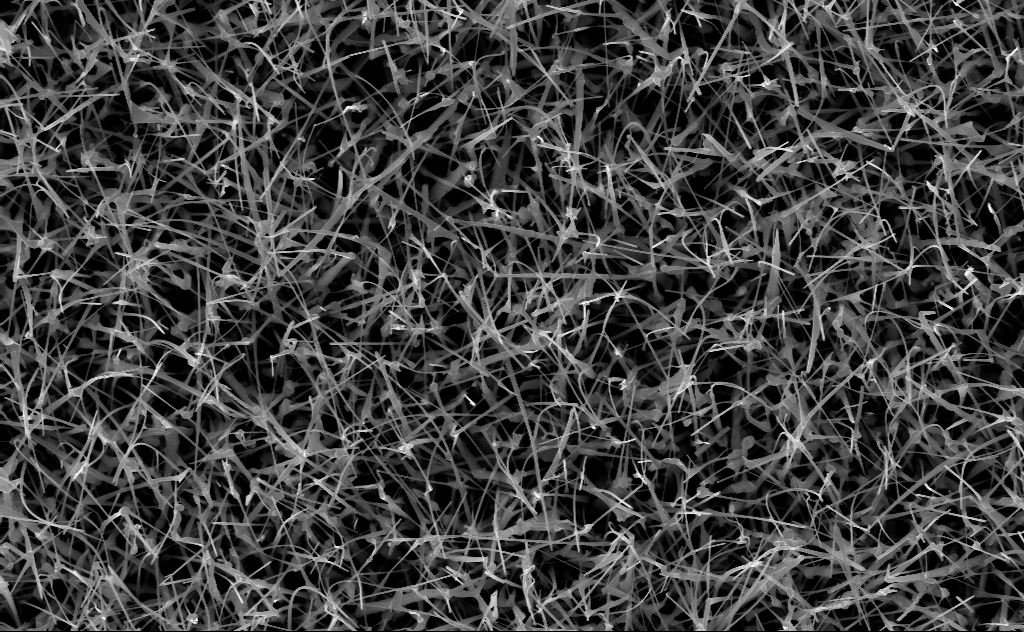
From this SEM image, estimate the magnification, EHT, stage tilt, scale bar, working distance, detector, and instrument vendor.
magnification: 20 K X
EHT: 10 kV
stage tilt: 0°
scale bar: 2000 nm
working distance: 6 mm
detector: InLens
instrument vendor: Zeiss